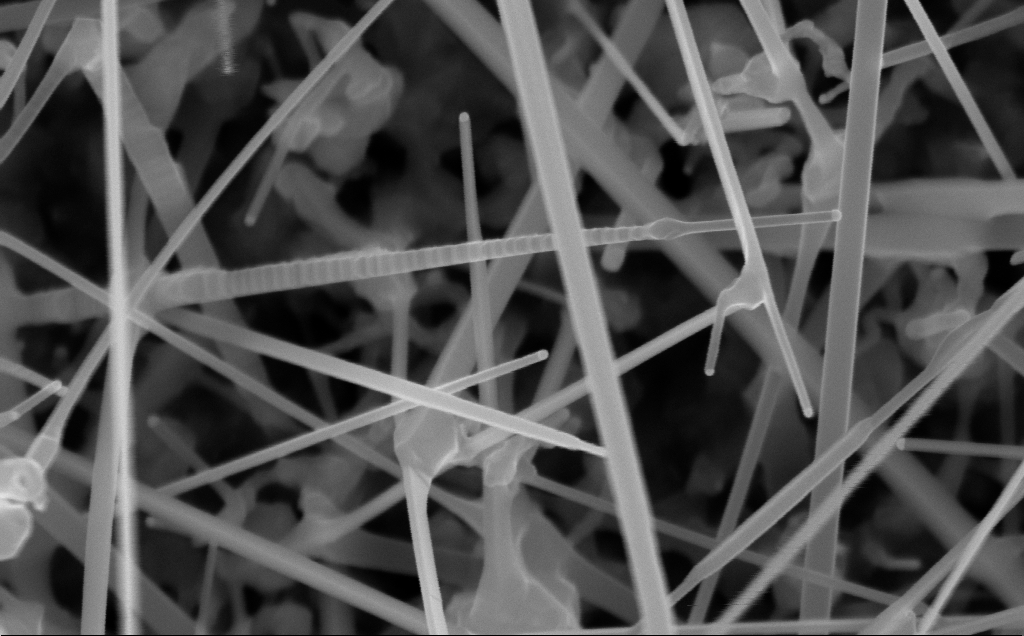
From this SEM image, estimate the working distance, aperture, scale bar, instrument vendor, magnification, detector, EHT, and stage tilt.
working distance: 8 mm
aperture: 30 µm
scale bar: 200 nm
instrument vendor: Zeiss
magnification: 129.06 K X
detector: InLens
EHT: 10 kV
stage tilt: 0°